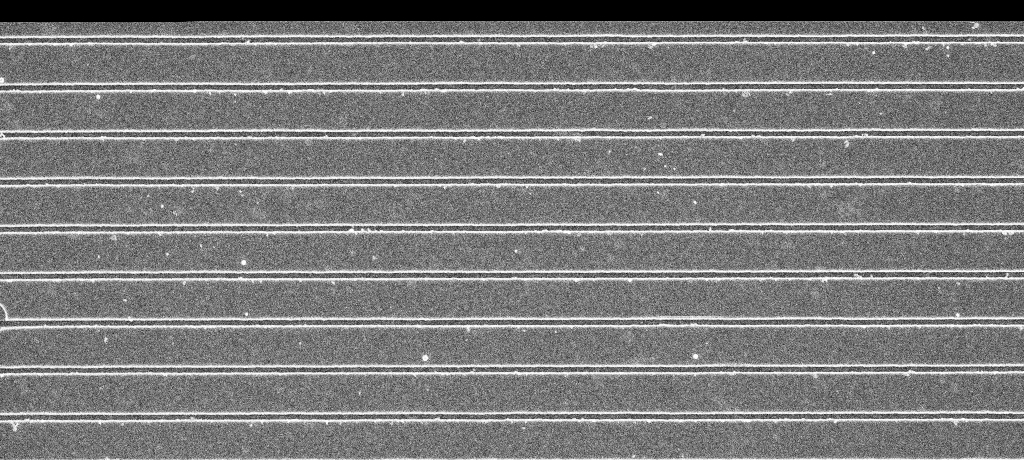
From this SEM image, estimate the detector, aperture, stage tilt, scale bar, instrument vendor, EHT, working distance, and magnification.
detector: InLens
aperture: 30 µm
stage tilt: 0°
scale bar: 2000 nm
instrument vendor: Zeiss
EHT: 5 kV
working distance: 5.4 mm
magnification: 19.28 K X